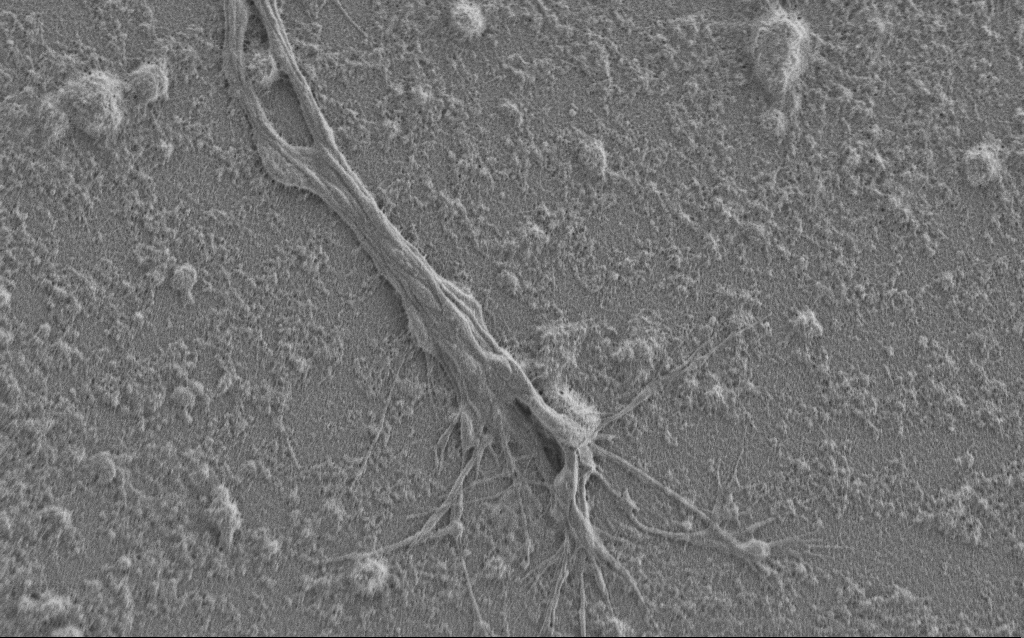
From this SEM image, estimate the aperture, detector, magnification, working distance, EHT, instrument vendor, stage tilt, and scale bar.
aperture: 30 µm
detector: SE2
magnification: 7.5 K X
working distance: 6 mm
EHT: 1 kV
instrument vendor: Zeiss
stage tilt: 0°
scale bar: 2000 nm